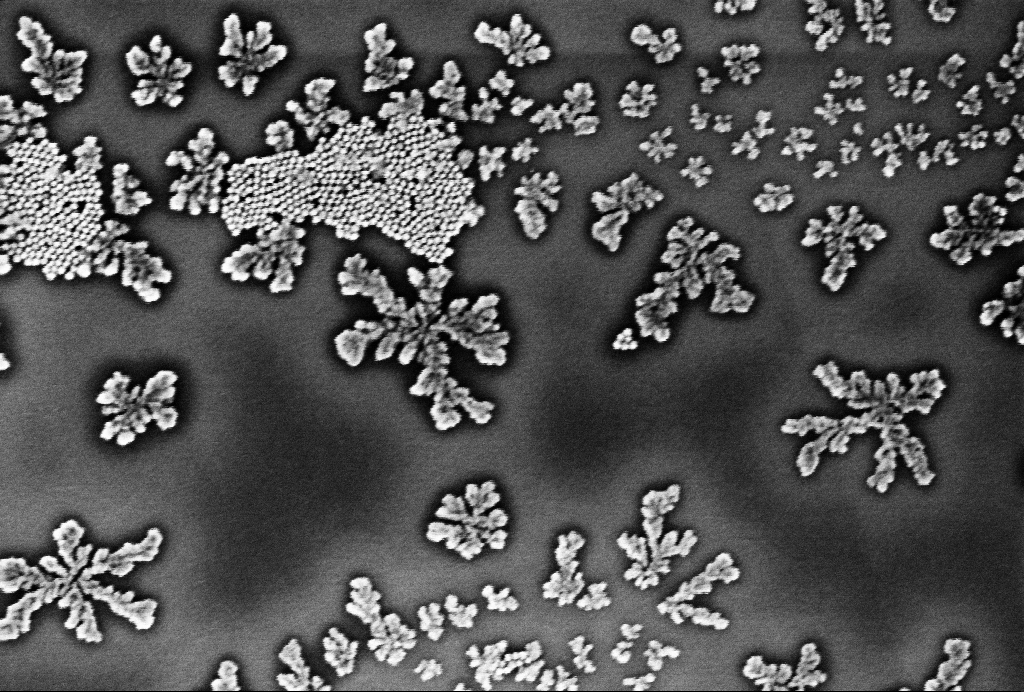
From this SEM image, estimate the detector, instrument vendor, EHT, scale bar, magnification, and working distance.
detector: InLens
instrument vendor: Zeiss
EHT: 1 kV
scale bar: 100 nm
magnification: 119.11 K X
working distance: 3.1 mm